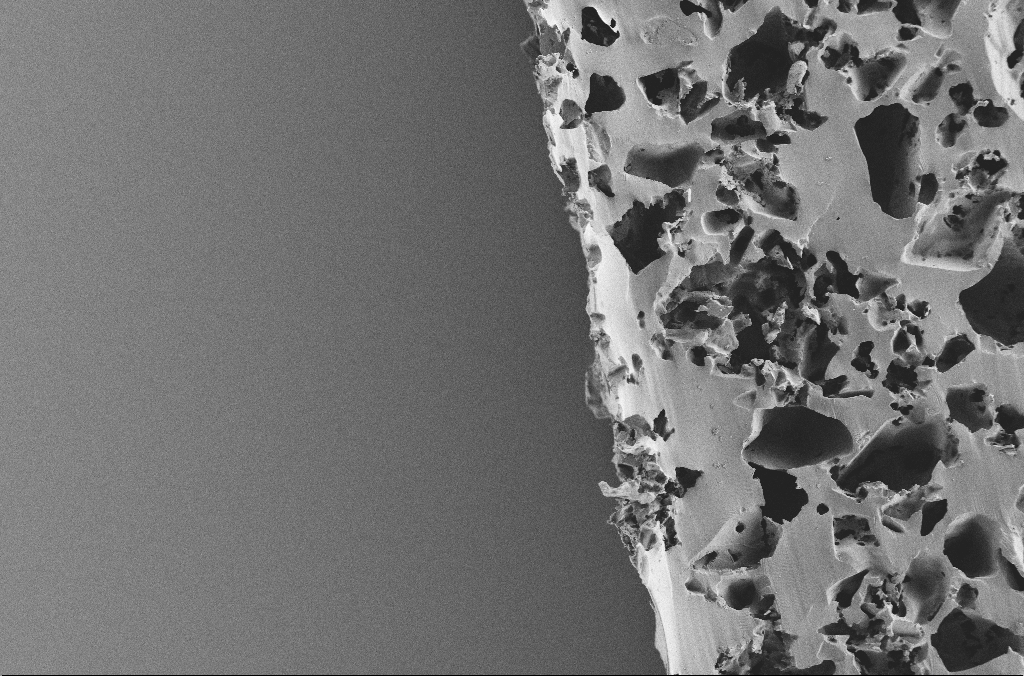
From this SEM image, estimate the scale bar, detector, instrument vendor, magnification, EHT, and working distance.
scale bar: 100000 nm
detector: SE2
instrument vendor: Zeiss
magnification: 0.25 K X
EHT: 2 kV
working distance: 3.1 mm